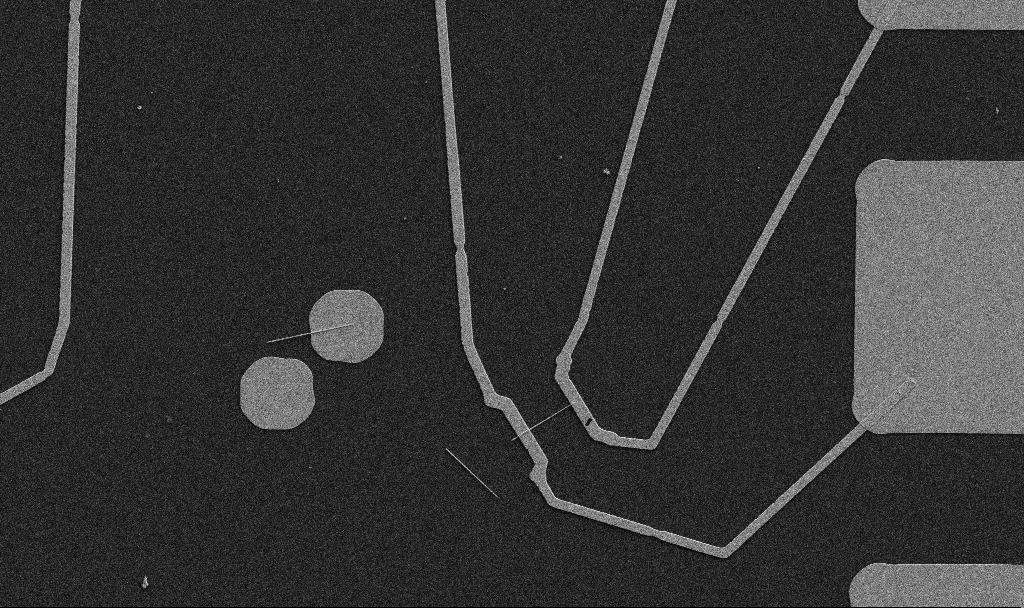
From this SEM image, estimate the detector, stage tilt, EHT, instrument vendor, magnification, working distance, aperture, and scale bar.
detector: SE2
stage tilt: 0°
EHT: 5 kV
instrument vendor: Zeiss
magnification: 5 K X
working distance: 10.7 mm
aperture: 30 µm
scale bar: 10000 nm